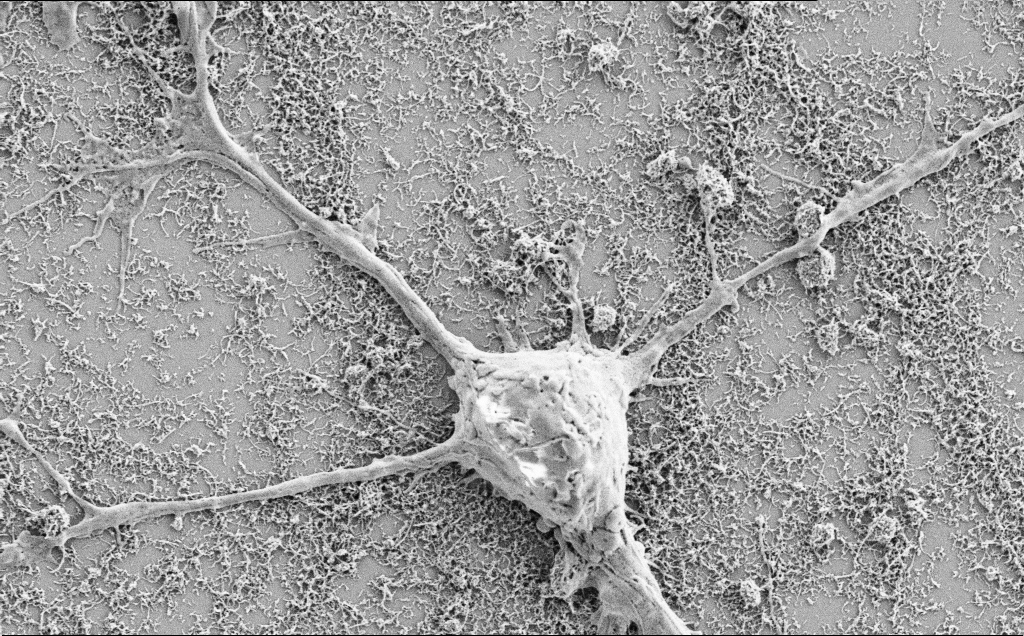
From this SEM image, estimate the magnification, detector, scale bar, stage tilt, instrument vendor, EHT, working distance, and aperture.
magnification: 10 K X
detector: SE2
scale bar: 2000 nm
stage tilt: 0°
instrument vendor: Zeiss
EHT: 2 kV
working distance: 7.1 mm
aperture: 30 µm